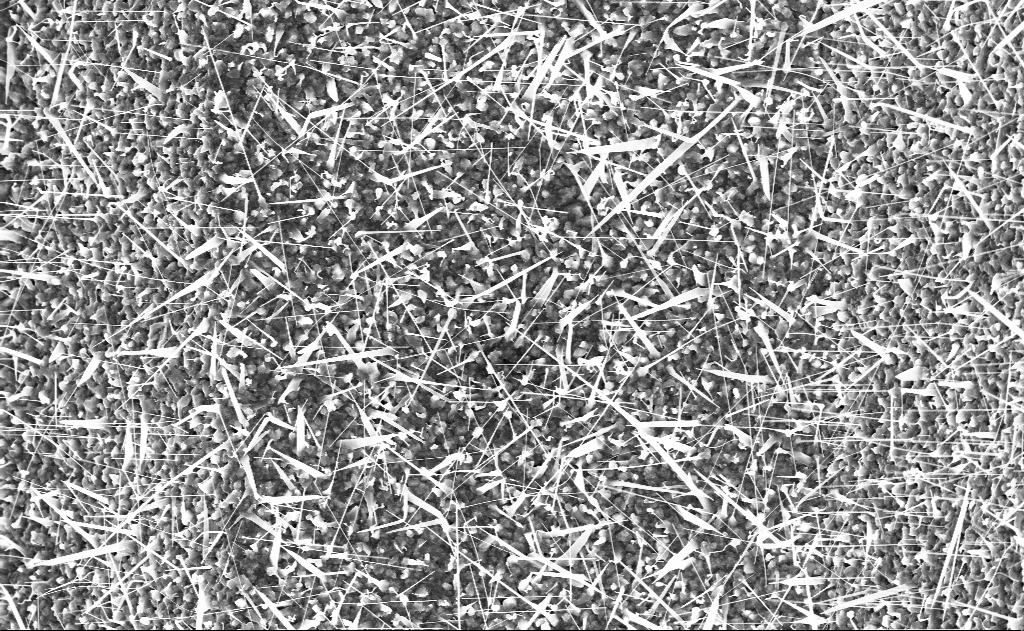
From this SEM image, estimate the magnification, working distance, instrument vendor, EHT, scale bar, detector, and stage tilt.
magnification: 10 K X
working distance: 9 mm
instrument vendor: Zeiss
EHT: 10 kV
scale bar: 2000 nm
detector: InLens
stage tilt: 0°